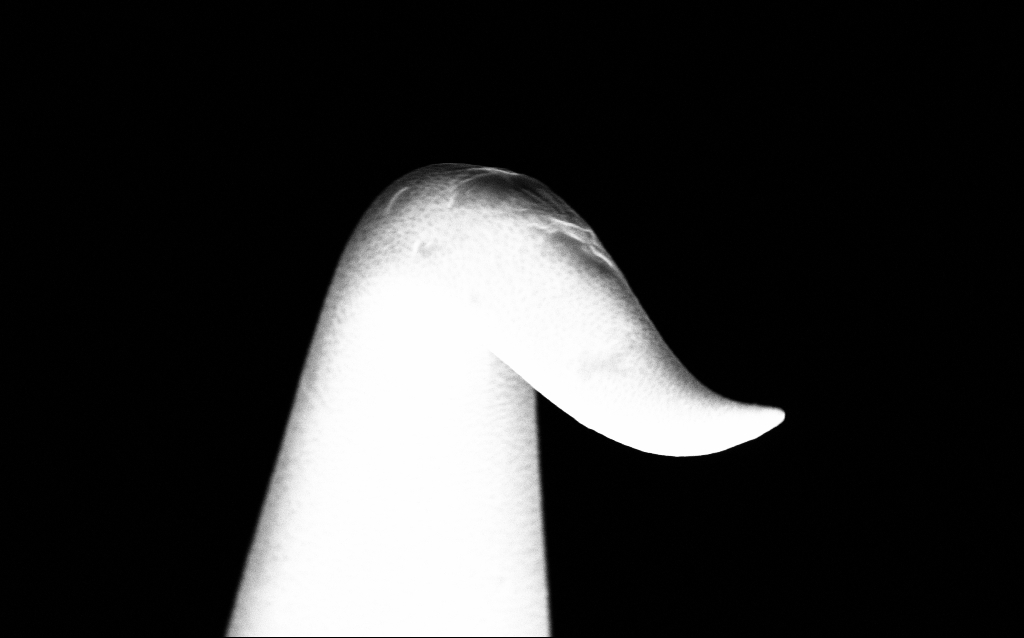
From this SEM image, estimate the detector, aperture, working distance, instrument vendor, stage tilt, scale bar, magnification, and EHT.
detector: InLens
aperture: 30 µm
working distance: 5.3 mm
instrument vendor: Zeiss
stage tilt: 45°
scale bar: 1000 nm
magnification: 54.28 K X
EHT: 10 kV